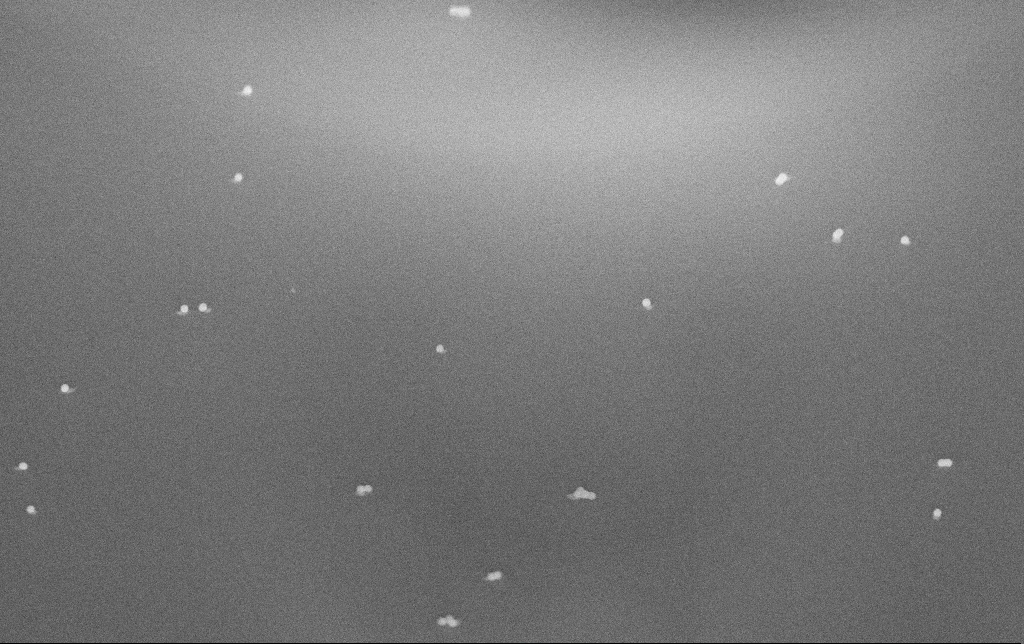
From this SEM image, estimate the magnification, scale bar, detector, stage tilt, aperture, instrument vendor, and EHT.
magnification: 50 K X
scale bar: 1000 nm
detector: InLens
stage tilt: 60°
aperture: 30 µm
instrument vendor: Zeiss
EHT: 10 kV